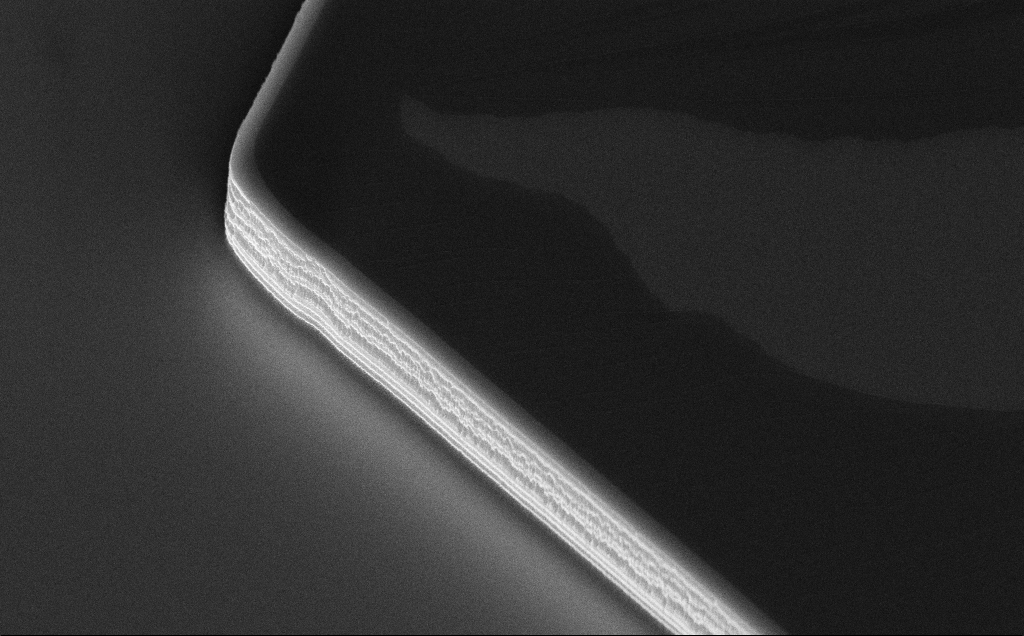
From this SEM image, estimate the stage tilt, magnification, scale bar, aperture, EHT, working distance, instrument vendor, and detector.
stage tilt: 50°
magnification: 11.78 K X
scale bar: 2000 nm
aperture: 30 µm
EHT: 10 kV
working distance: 10 mm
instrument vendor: Zeiss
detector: InLens